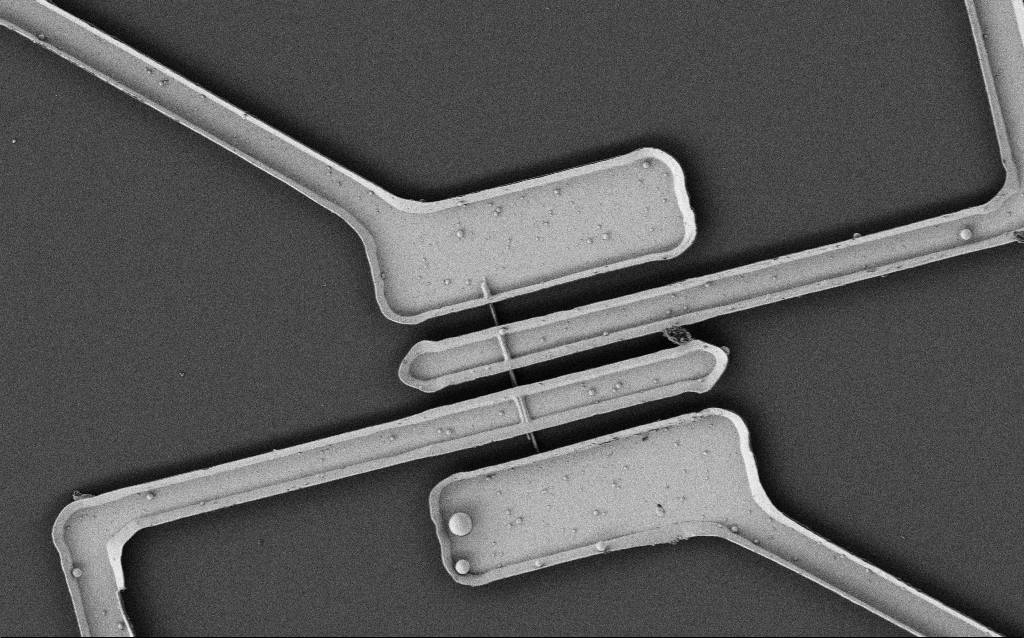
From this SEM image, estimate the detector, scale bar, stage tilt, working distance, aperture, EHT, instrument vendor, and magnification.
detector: SE2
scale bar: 10000 nm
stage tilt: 0°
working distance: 7.7 mm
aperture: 30 µm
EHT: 2 kV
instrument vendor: Zeiss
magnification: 3.9 K X